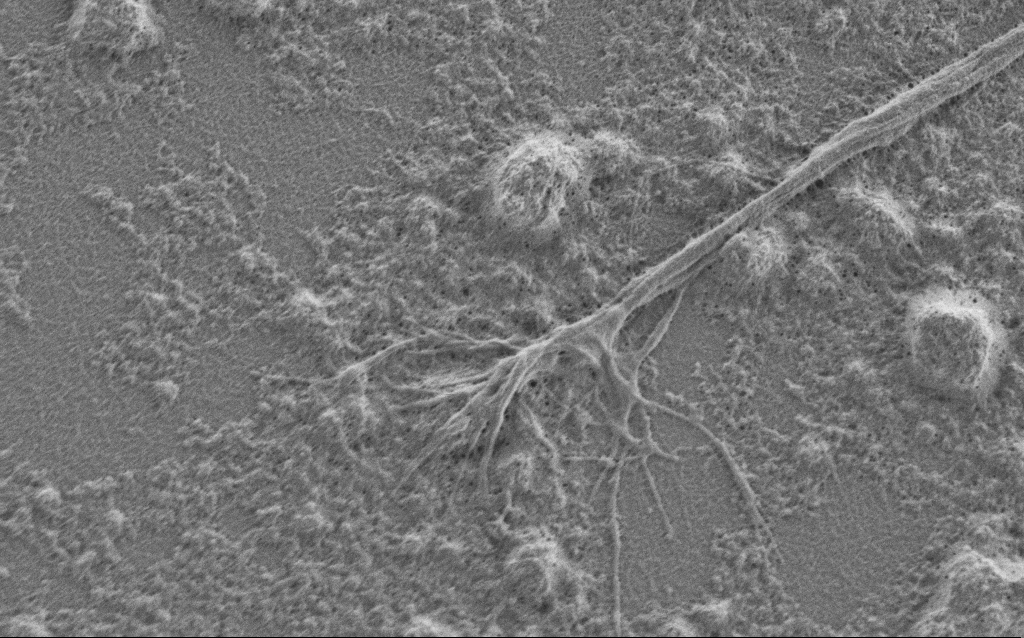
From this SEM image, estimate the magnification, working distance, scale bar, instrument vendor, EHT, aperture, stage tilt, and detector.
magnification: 10 K X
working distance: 6 mm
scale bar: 2000 nm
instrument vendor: Zeiss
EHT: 1 kV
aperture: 30 µm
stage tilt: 0°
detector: SE2